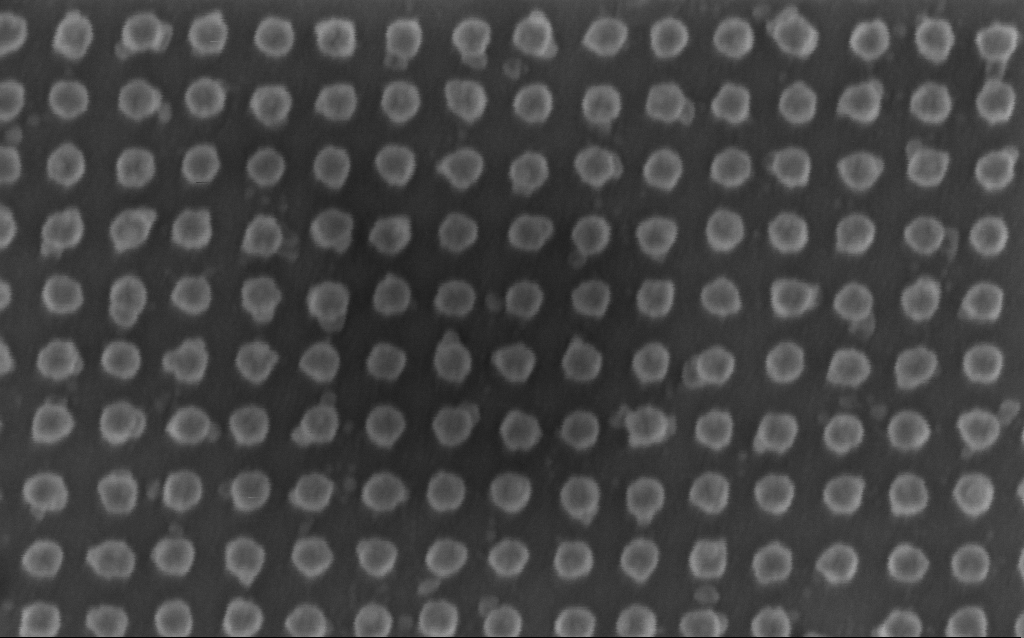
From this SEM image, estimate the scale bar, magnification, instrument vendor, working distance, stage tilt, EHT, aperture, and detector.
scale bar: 200 nm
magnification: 171.72 K X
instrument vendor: Zeiss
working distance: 6 mm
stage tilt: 0°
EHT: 1.5 kV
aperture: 30 µm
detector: InLens